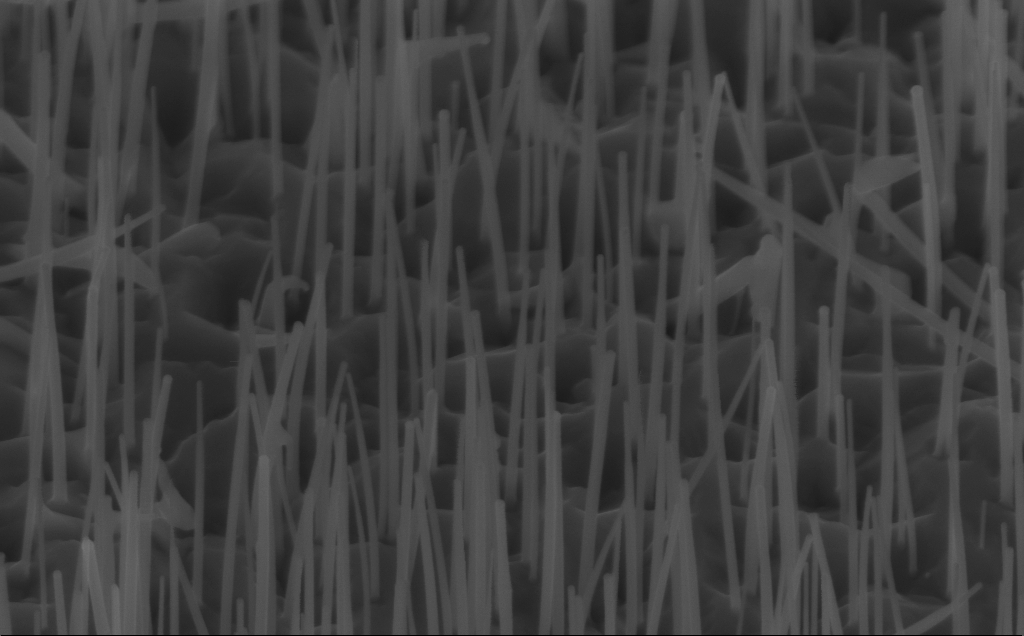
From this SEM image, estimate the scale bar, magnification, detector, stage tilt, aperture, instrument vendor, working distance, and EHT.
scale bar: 200 nm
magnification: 80 K X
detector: InLens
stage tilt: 45°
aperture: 30 µm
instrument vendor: Zeiss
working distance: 5 mm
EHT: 10 kV